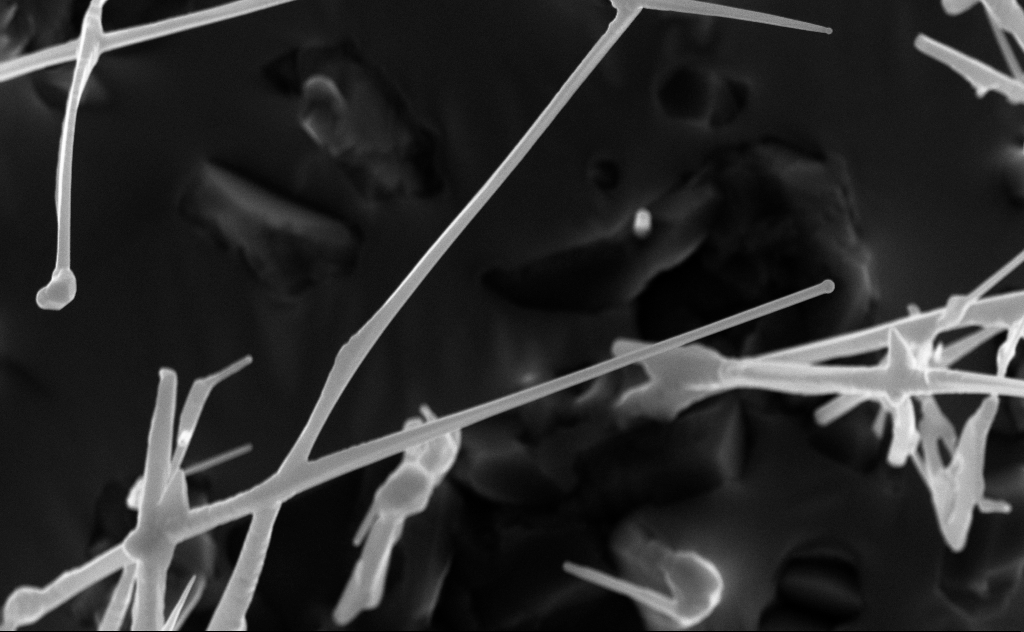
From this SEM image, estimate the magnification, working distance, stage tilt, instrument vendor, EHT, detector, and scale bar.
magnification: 40 K X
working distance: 6 mm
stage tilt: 0°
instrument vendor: Zeiss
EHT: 10 kV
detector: InLens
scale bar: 1000 nm